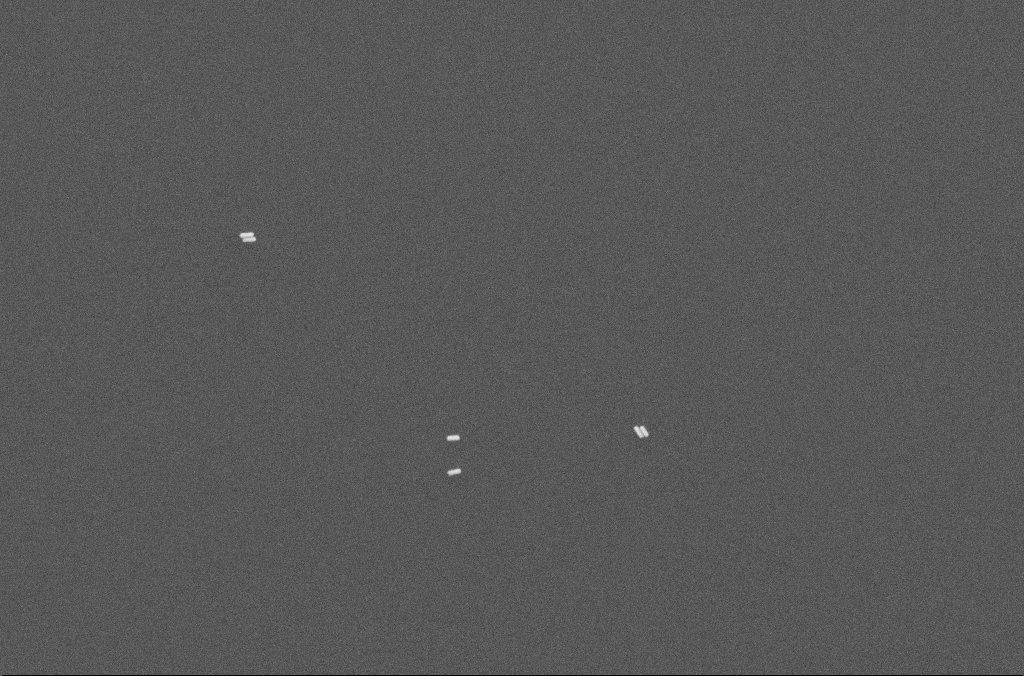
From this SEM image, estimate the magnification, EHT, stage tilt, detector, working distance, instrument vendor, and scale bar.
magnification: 71.61 K X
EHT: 10 kV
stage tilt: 0°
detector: SE2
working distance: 2.5 mm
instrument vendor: Zeiss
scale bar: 200 nm